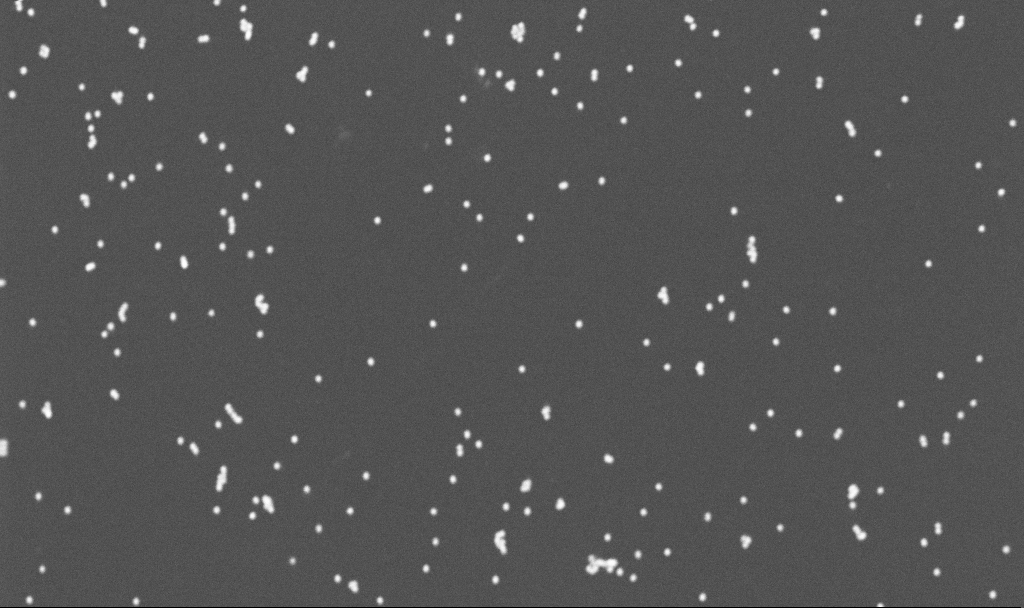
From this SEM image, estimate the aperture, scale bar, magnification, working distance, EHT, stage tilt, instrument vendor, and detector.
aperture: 30 µm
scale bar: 1000 nm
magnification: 70 K X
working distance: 3.3 mm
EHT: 10 kV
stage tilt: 0°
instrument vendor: Zeiss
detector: InLens